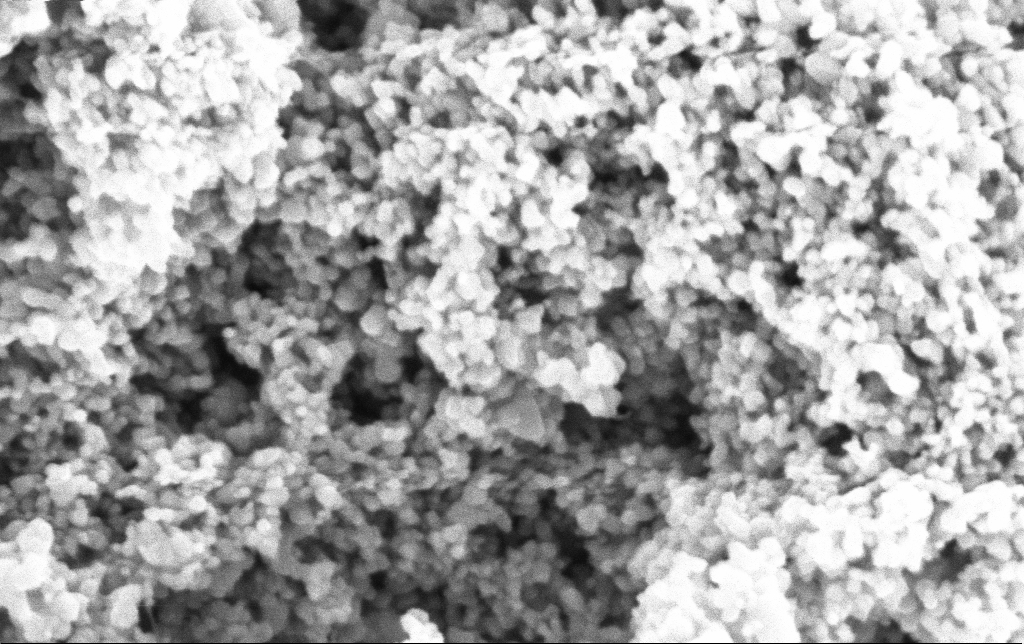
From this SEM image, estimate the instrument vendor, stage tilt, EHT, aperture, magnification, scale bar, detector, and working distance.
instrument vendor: Zeiss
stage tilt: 0°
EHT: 3 kV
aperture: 30 µm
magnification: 204.67 K X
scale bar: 200 nm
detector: InLens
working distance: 2.8 mm